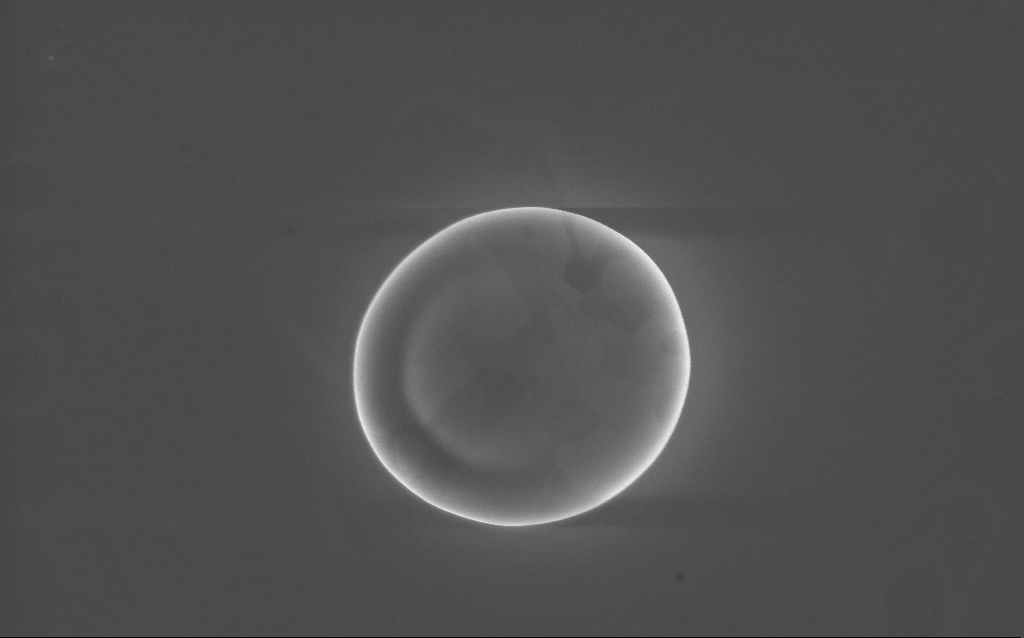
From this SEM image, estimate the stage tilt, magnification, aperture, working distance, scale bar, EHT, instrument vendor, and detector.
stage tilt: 0°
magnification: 36.42 K X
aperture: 30 µm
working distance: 2 mm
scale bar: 1000 nm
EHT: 10 kV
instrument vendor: Zeiss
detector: InLens